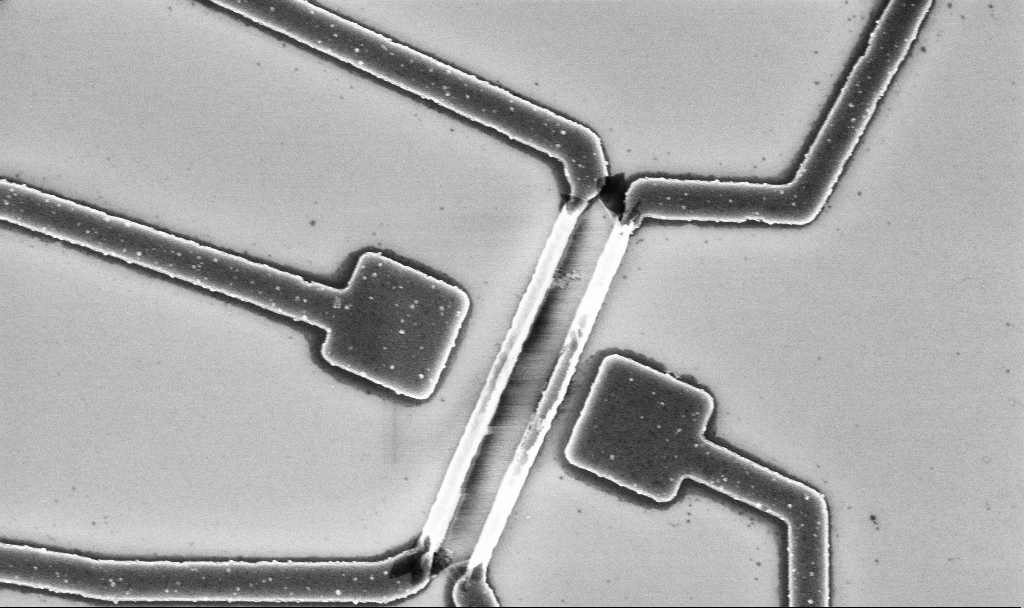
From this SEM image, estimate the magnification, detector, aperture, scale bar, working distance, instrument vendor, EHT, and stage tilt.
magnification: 20 K X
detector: InLens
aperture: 30 µm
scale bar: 2000 nm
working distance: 10.7 mm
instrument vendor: Zeiss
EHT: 5 kV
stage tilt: -0°